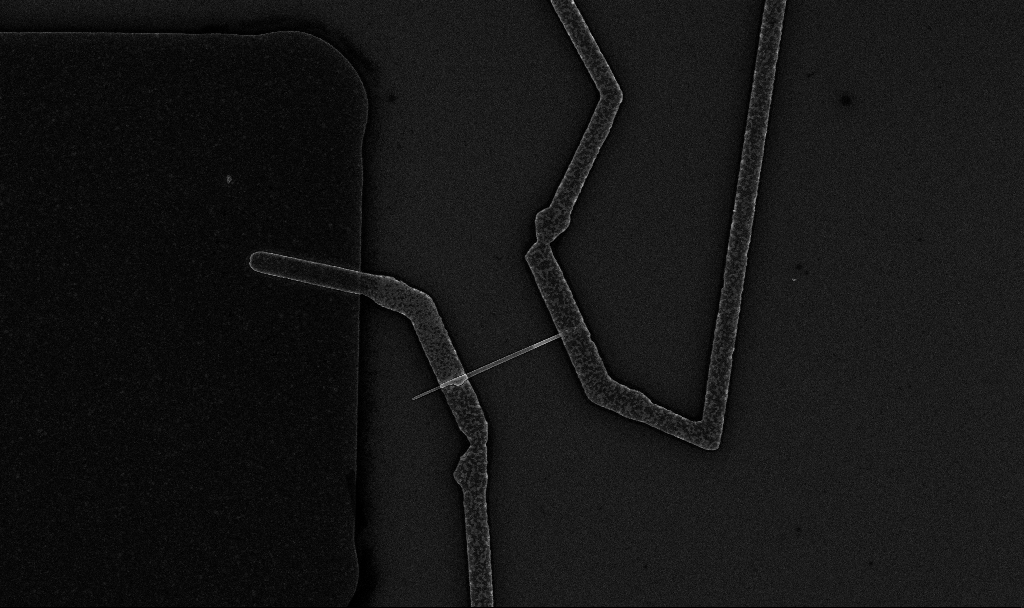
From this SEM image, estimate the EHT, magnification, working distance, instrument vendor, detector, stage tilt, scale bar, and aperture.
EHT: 10 kV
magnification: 11.13 K X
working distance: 6.7 mm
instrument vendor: Zeiss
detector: InLens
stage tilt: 0°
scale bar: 2000 nm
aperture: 30 µm